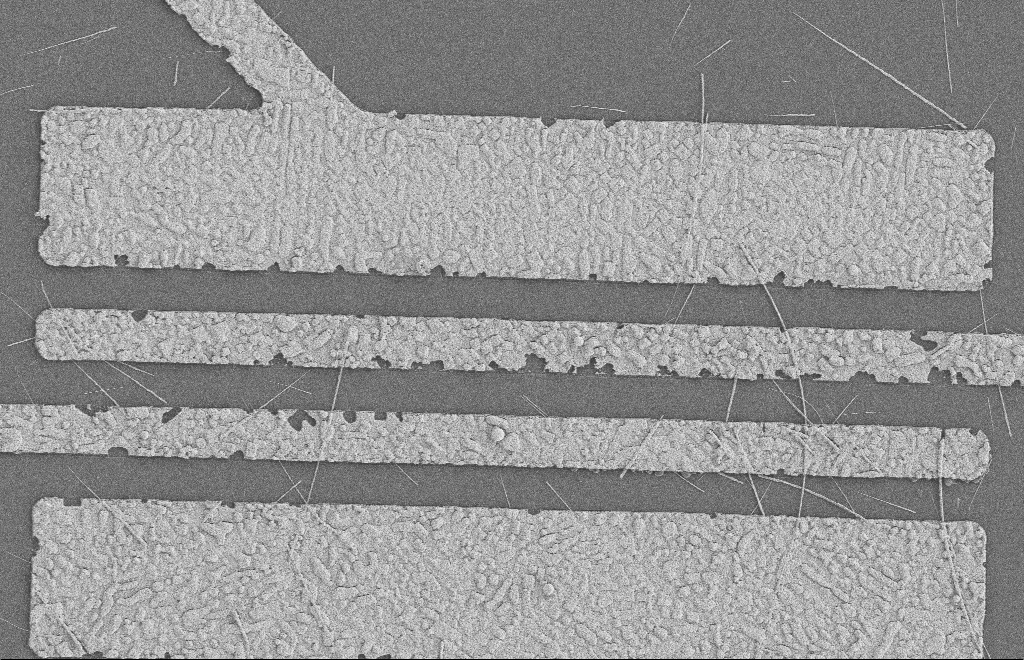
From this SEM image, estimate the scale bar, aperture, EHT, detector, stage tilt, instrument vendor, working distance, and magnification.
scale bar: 2000 nm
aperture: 20 µm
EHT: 2 kV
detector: SE2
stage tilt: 0°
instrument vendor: Zeiss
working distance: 8 mm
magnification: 5.75 K X